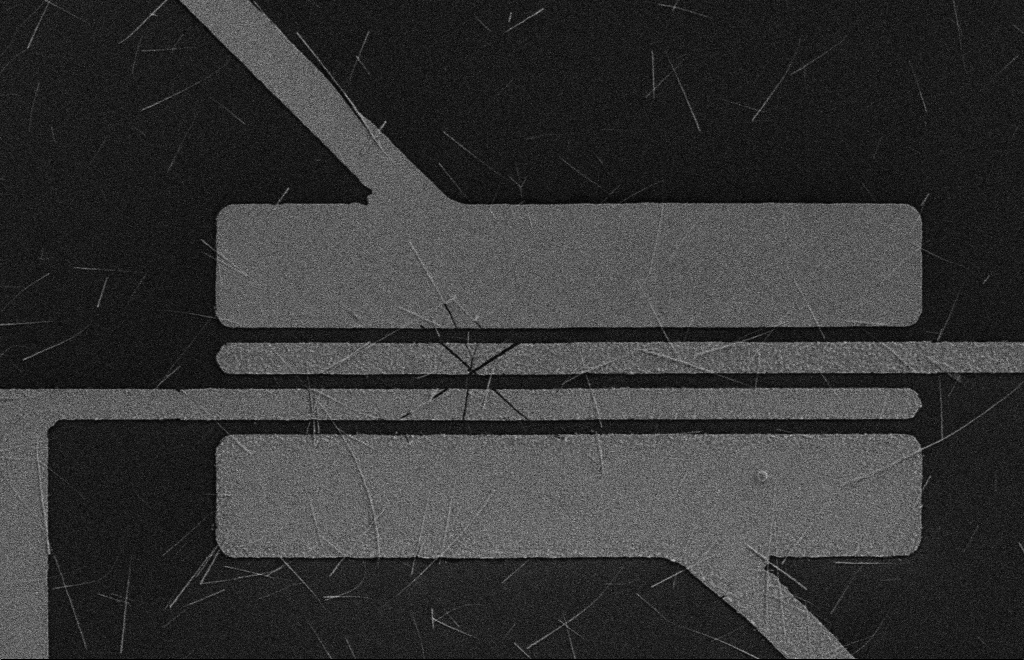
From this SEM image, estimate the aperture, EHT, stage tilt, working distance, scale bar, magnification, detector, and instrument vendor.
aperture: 10 µm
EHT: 5 kV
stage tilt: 0°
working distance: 16 mm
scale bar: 10000 nm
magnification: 4.26 K X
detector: SE2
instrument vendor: Zeiss